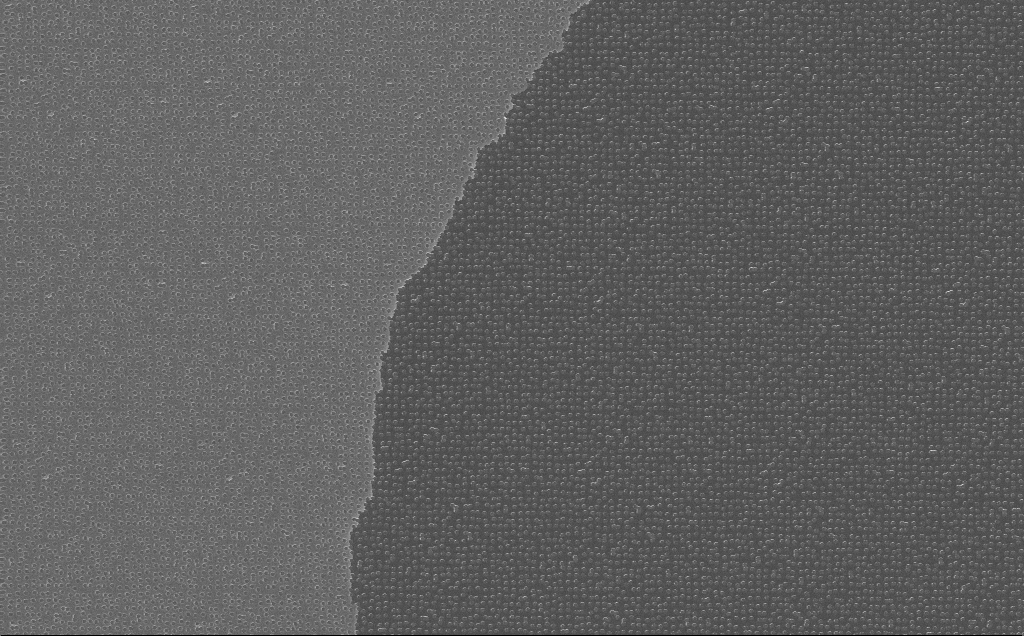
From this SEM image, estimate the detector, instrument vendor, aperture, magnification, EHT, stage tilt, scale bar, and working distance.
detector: InLens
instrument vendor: Zeiss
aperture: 30 µm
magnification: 6.48 K X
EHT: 10 kV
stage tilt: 0°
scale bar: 10000 nm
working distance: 7 mm